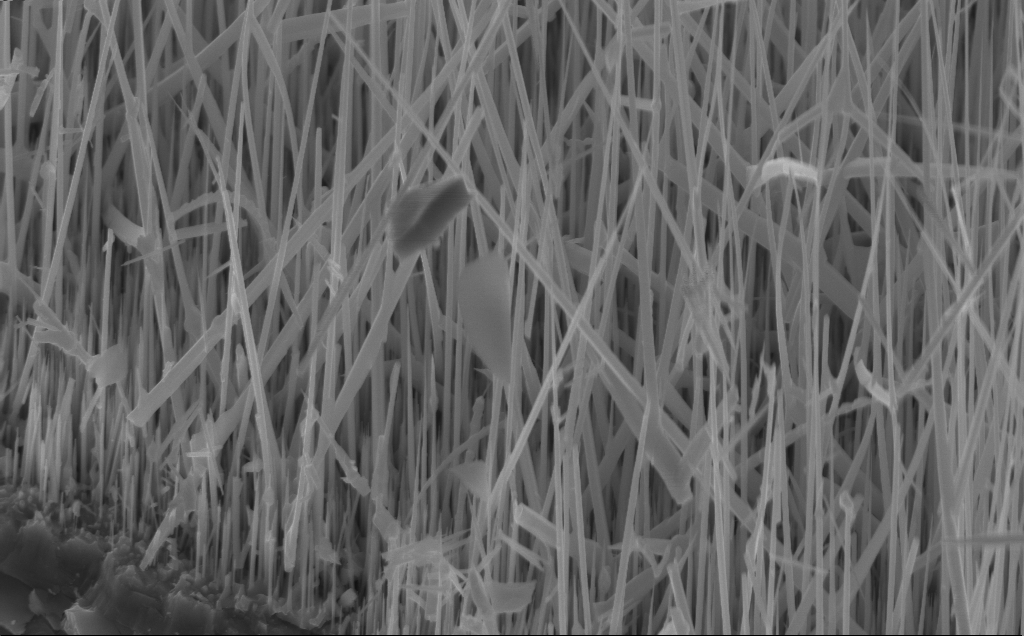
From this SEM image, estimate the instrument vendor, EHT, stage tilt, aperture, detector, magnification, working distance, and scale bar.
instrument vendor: Zeiss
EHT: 10 kV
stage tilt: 45°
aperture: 30 µm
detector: InLens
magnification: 20 K X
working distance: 5 mm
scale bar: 2000 nm